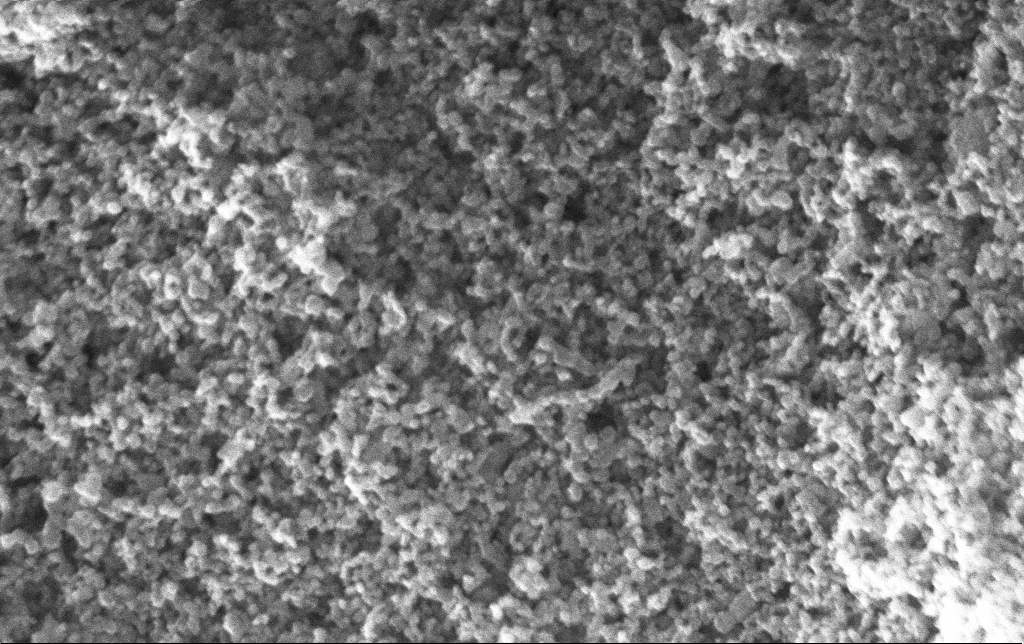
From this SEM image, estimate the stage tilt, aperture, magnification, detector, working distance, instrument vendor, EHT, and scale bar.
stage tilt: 0°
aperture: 30 µm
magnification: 135 K X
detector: InLens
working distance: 2.6 mm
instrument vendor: Zeiss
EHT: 10 kV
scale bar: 200 nm